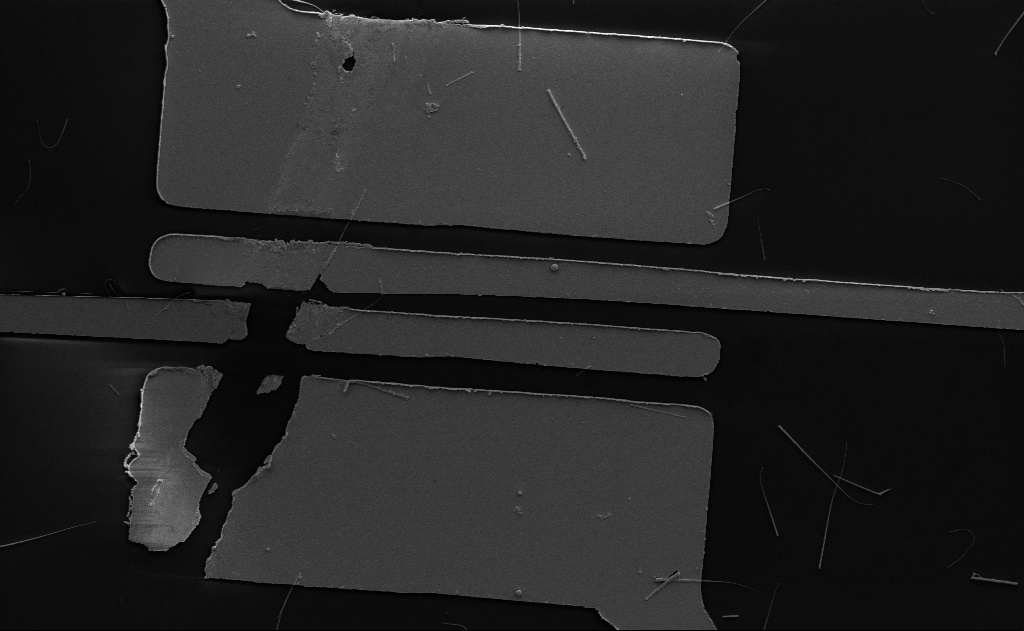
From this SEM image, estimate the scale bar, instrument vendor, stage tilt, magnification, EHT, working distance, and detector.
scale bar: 10000 nm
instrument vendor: Zeiss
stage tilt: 0°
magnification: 6.92 K X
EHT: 5 kV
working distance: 15 mm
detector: SE2